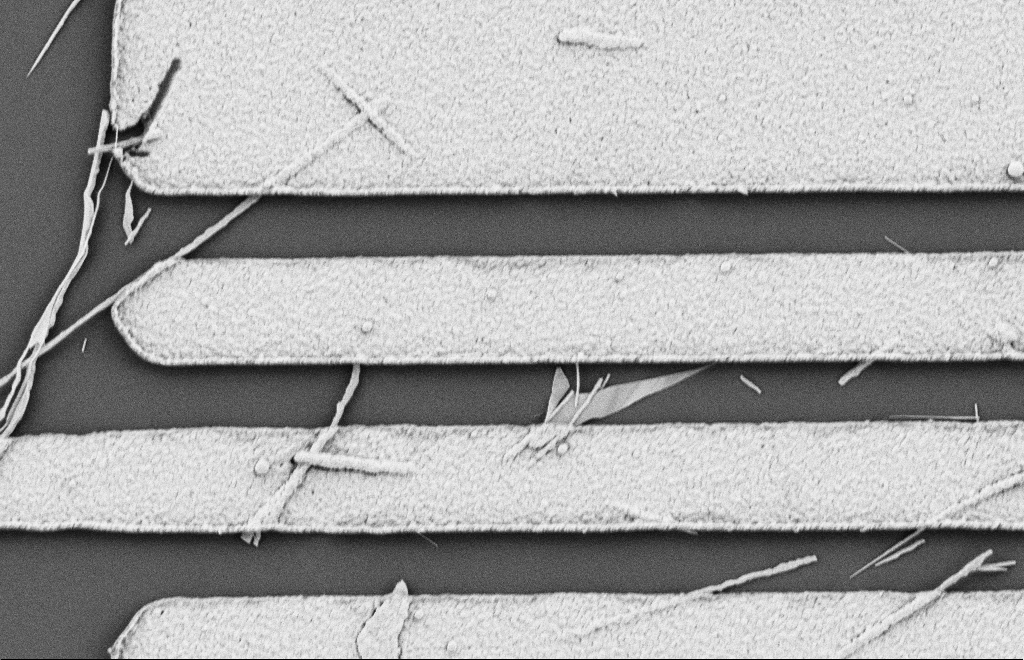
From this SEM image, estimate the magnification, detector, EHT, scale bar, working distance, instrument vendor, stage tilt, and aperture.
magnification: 15.37 K X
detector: SE2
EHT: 2 kV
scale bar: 2000 nm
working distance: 10 mm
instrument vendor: Zeiss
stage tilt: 0°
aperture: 20 µm